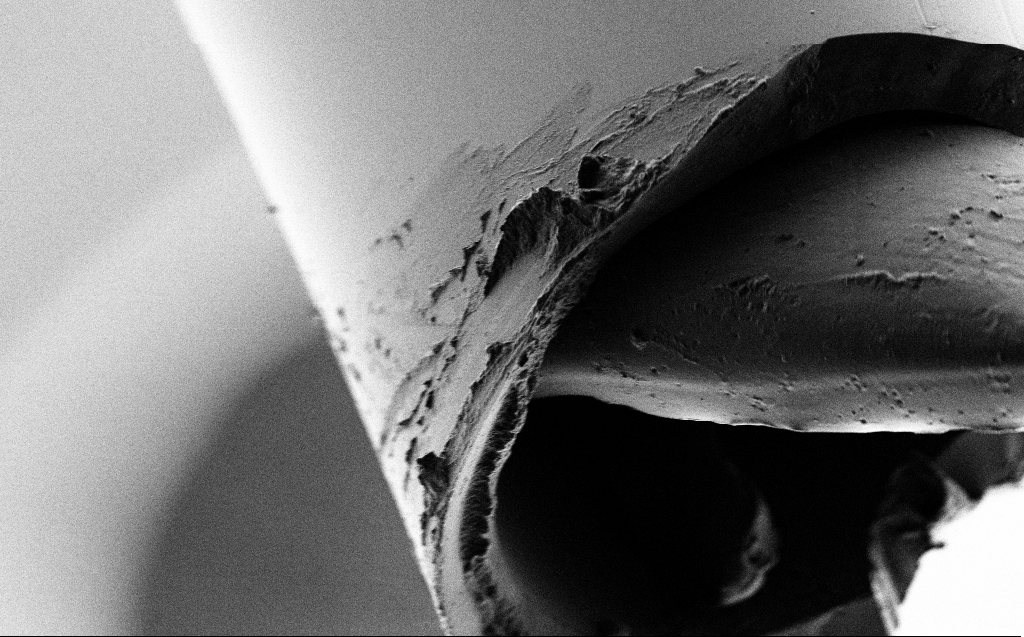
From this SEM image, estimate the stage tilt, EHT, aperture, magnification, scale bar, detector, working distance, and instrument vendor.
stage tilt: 45°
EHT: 1 kV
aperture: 30 µm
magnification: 3.95 K X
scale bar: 10000 nm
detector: SE2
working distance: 3 mm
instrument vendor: Zeiss